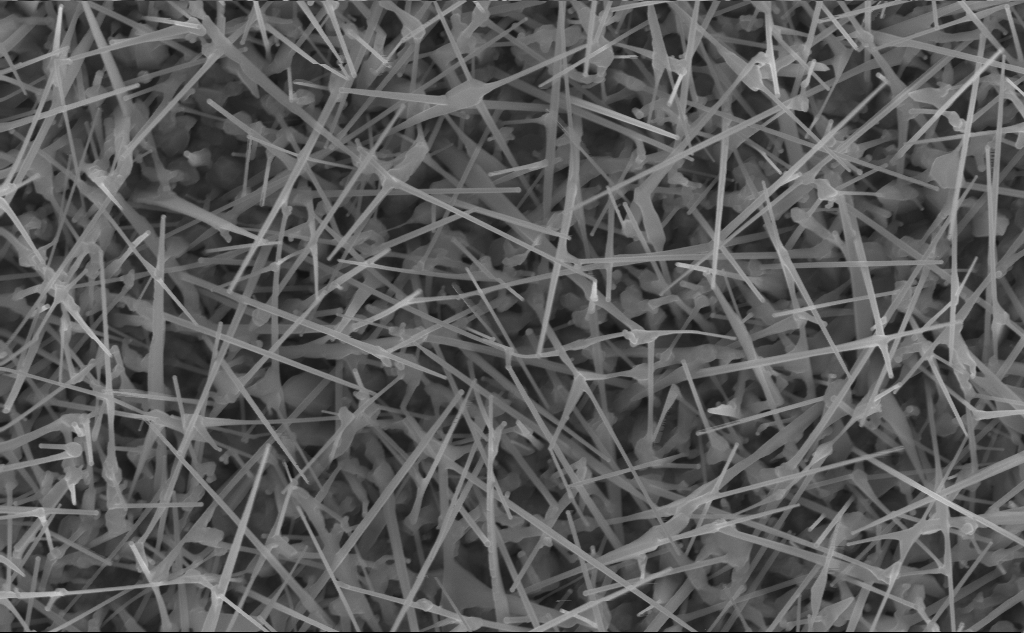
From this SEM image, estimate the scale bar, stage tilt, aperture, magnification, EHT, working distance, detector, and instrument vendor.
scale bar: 1000 nm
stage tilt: -0.2°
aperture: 30 µm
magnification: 40 K X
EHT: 10 kV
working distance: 7 mm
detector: InLens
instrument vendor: Zeiss